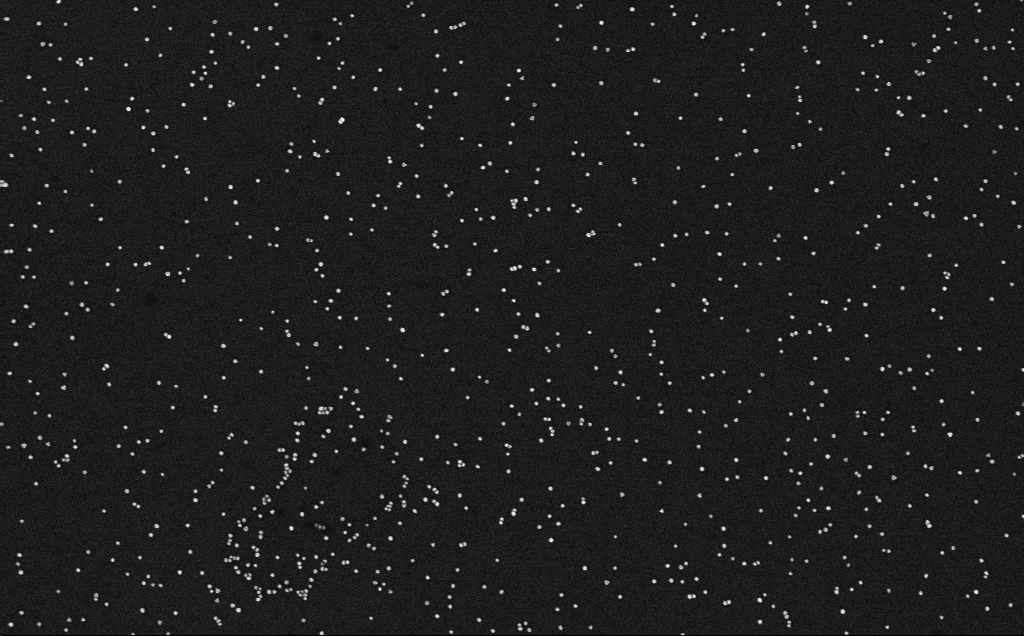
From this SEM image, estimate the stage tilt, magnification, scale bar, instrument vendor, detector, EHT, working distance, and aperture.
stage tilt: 0°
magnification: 100 K X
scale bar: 200 nm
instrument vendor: Zeiss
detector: InLens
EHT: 10 kV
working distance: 3.2 mm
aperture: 30 µm